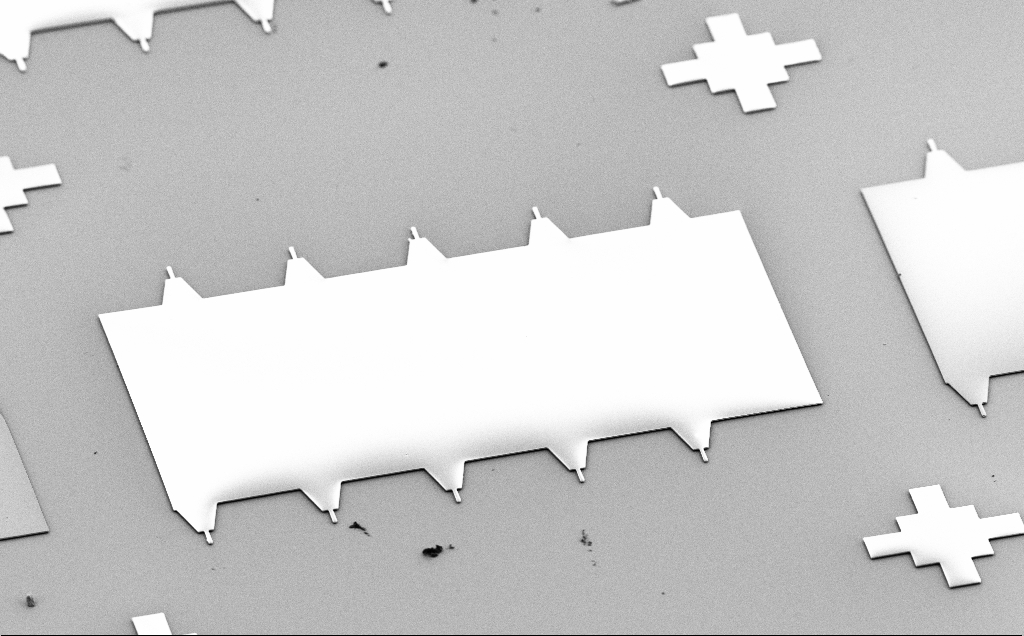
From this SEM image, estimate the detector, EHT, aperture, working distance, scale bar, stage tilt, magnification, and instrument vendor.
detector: SE2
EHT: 5 kV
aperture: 30 µm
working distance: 10 mm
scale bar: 100000 nm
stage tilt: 50°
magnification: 0.292 K X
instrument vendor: Zeiss